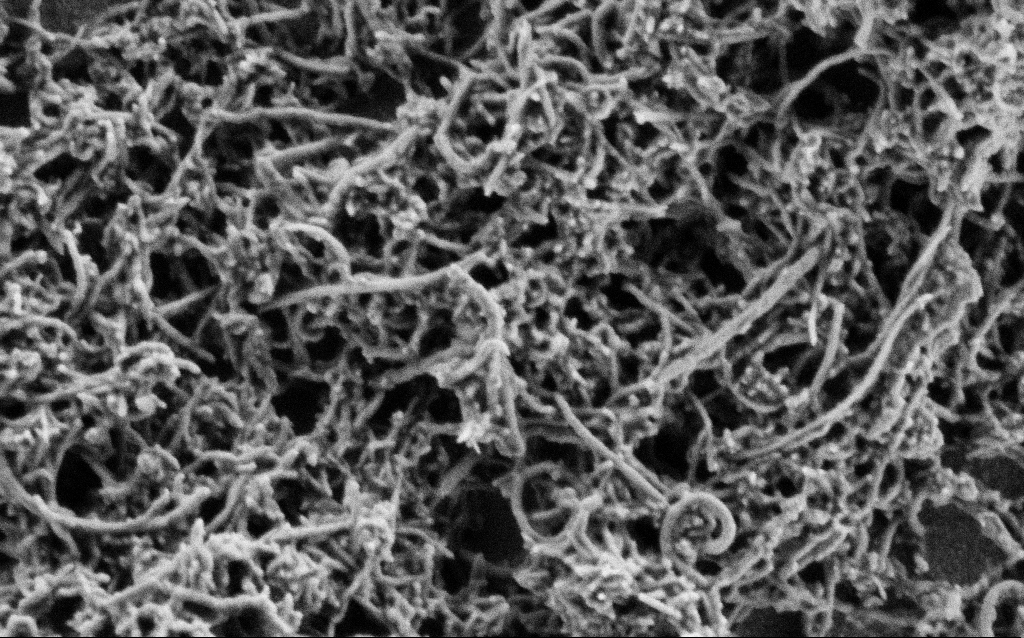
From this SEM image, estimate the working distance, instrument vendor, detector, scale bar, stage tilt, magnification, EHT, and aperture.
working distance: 4 mm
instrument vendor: Zeiss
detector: SE2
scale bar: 200 nm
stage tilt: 0°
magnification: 100 K X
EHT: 1 kV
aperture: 30 µm